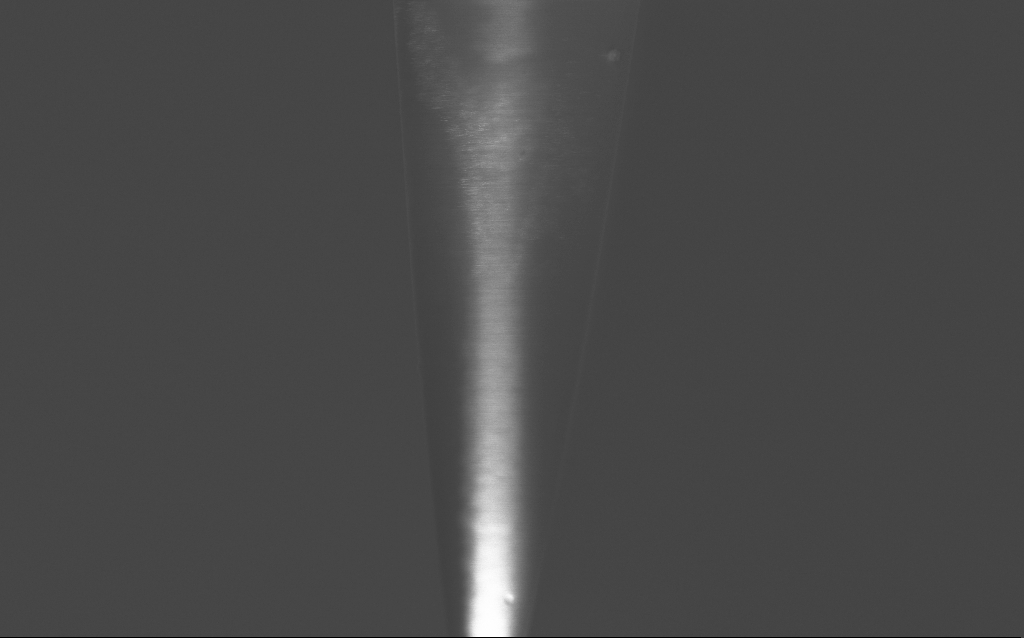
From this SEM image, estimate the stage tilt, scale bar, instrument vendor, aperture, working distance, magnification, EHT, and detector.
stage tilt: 45°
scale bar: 2000 nm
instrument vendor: Zeiss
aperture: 30 µm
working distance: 6 mm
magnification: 28.28 K X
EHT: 2 kV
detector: InLens